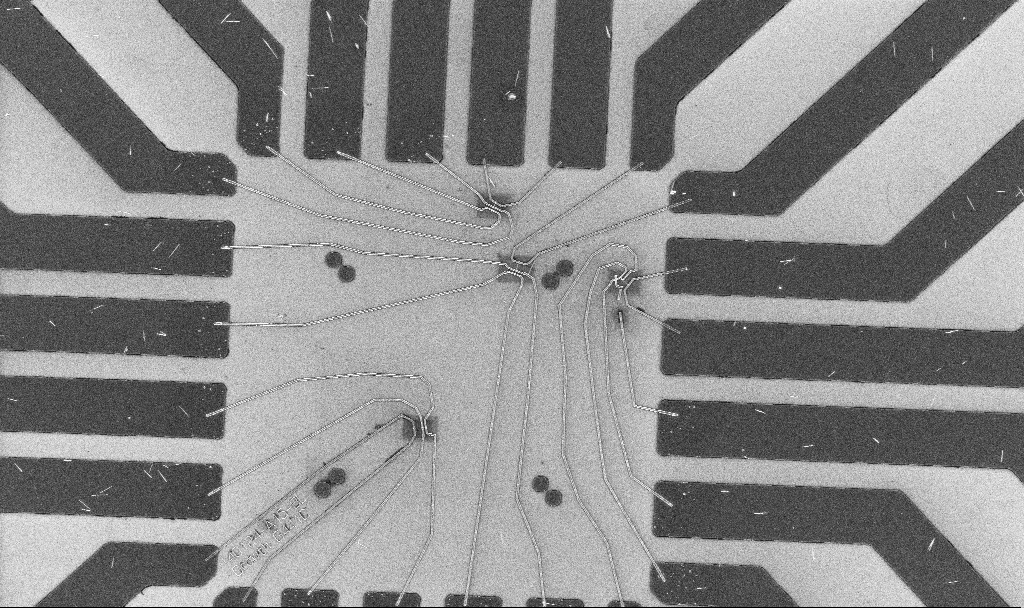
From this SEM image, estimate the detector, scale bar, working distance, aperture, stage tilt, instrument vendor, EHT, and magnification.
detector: InLens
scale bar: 20000 nm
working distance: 7.7 mm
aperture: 30 µm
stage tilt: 0°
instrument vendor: Zeiss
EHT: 10 kV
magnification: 1 K X